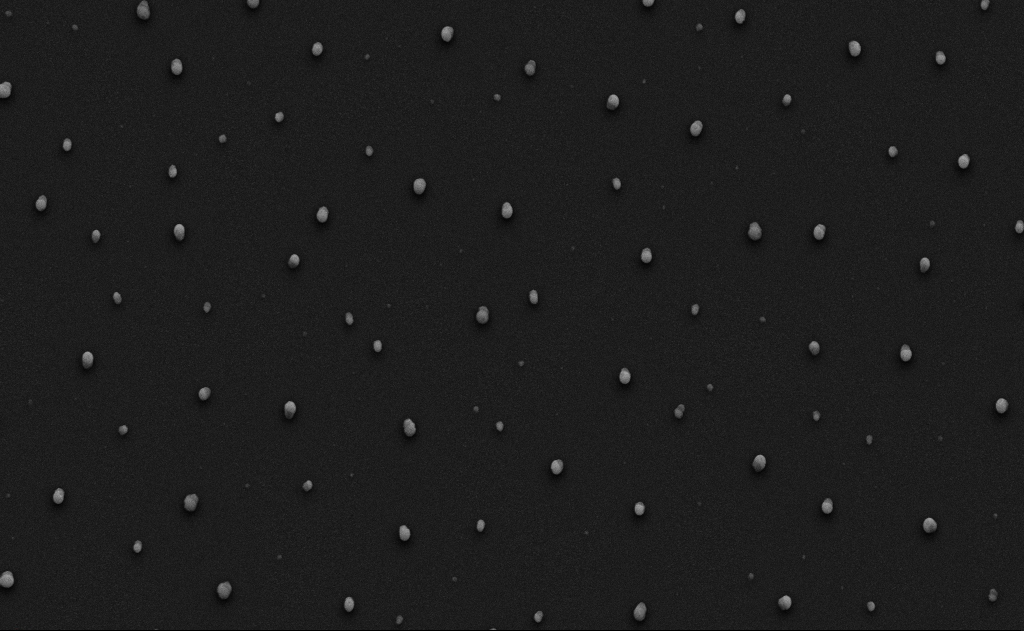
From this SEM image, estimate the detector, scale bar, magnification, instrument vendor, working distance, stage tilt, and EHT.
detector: SE2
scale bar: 2000 nm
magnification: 10 K X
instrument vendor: Zeiss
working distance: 9 mm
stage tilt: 0°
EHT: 10 kV